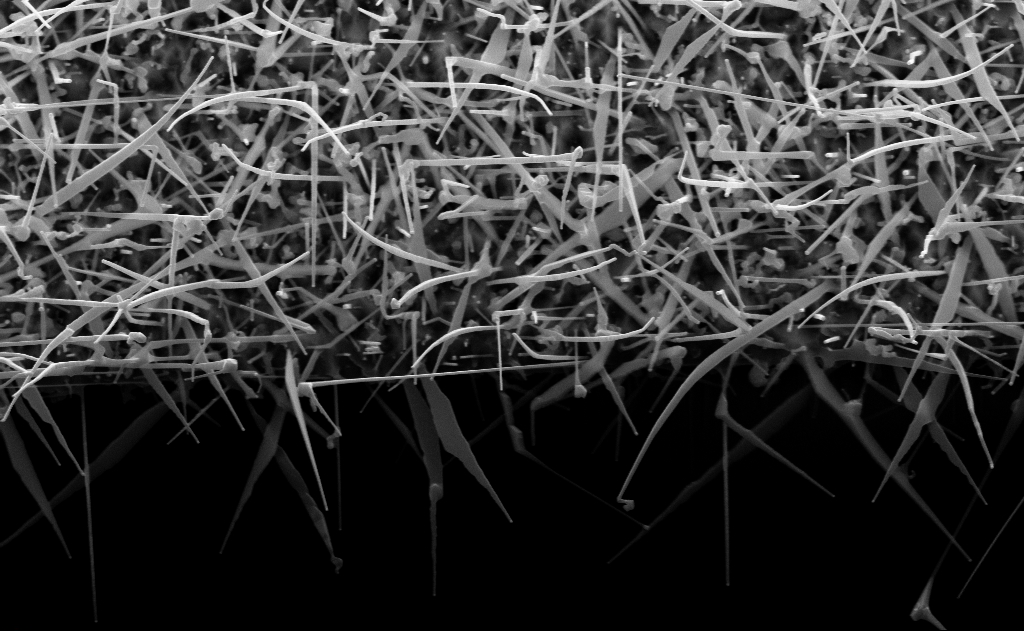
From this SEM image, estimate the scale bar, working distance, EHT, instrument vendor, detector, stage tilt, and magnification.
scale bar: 2000 nm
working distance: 11 mm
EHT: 10 kV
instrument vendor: Zeiss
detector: InLens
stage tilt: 0°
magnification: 30 K X